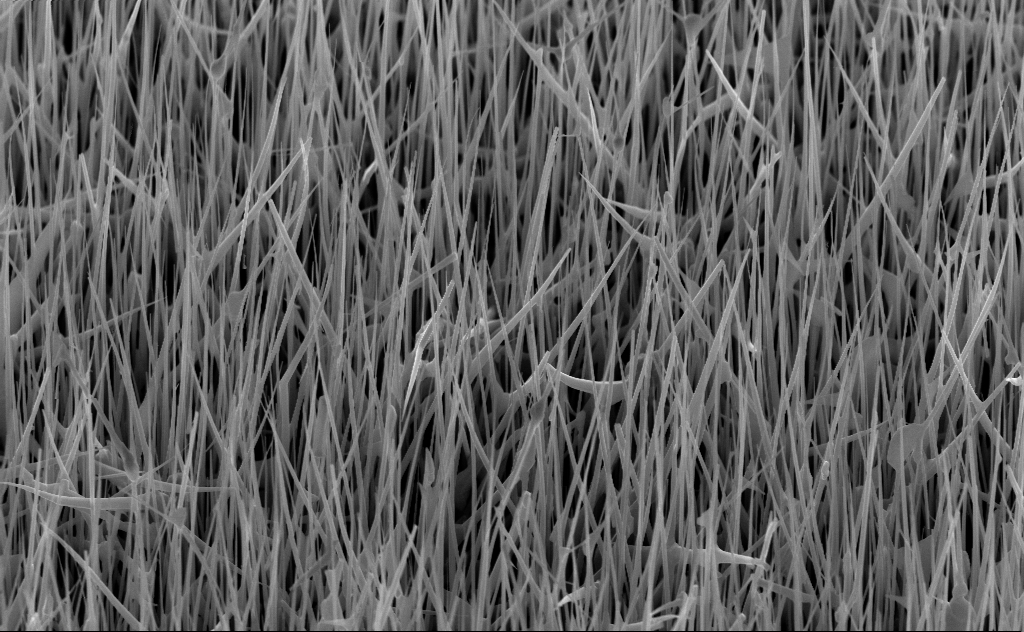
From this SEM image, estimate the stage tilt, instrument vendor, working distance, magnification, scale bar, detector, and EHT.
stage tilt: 45°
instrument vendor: Zeiss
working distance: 6 mm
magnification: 20 K X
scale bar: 1000 nm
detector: InLens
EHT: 10 kV